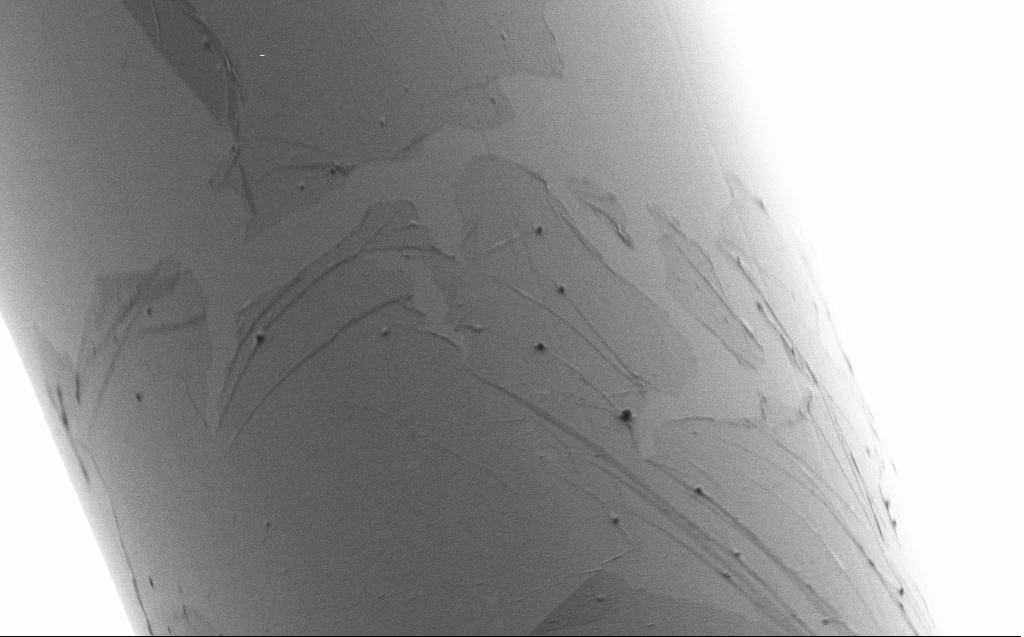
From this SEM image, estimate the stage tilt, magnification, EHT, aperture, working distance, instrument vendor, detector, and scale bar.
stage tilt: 45°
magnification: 10 K X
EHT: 1 kV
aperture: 30 µm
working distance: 4 mm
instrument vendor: Zeiss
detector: SE2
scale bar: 2000 nm